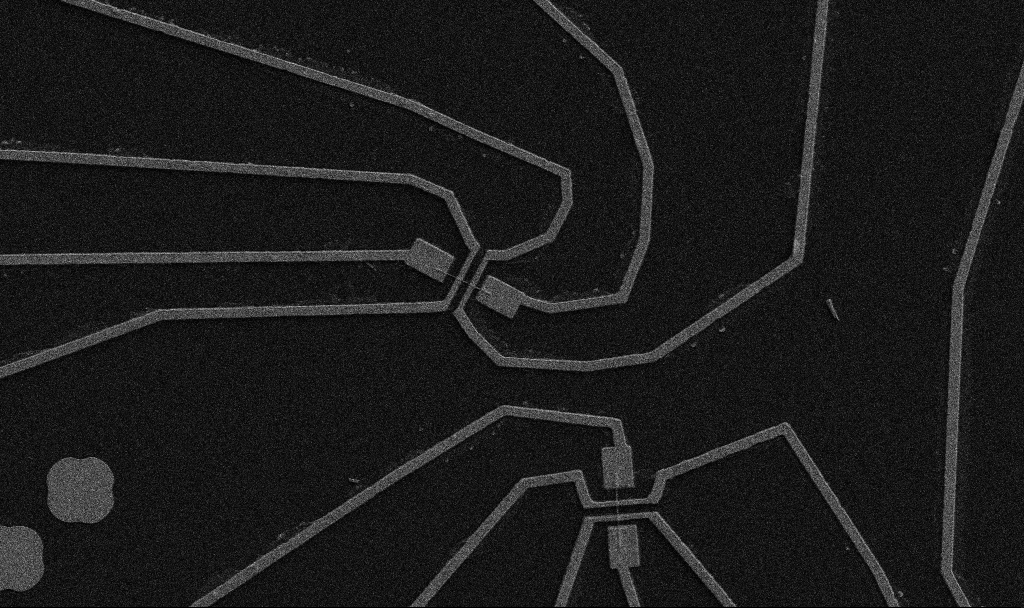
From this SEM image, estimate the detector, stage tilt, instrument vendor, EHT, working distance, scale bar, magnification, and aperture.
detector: SE2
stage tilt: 0°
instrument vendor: Zeiss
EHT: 5 kV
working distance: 10.7 mm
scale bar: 10000 nm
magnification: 5 K X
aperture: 30 µm